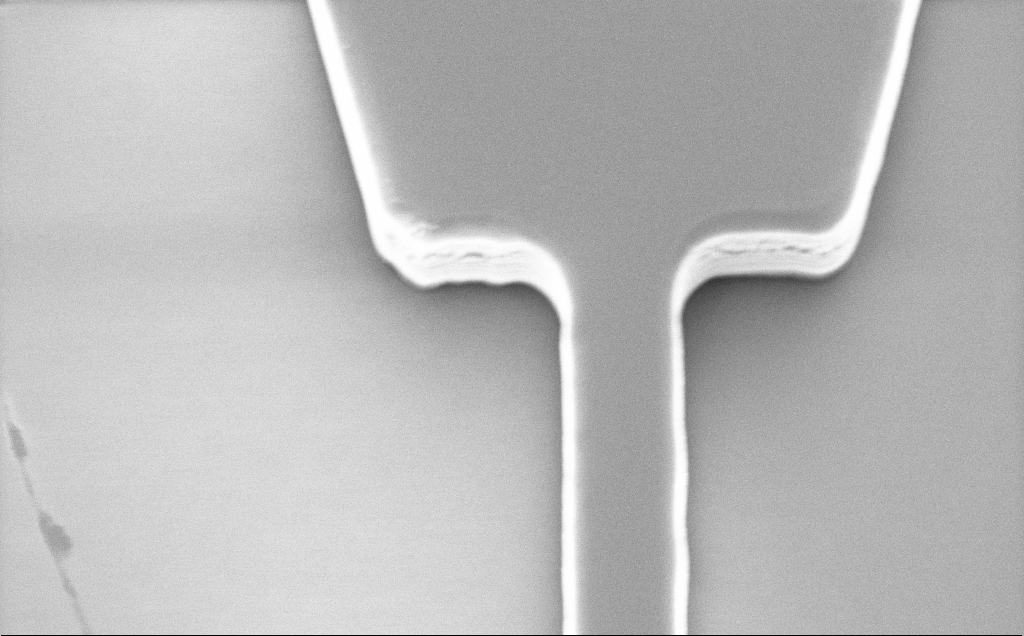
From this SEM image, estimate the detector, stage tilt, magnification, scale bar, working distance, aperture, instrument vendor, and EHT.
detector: SE2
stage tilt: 26.1°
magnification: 8.64 K X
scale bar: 2000 nm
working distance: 9 mm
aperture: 30 µm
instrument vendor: Zeiss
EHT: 5 kV